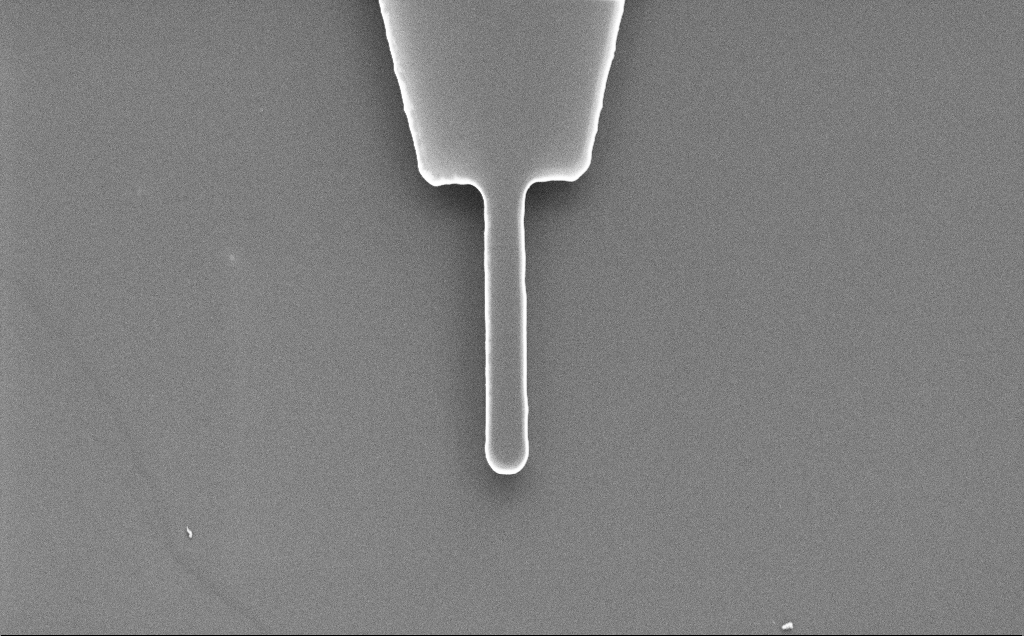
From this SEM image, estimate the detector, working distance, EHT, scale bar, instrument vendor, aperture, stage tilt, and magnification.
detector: SE2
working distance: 7 mm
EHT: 5 kV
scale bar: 10000 nm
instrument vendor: Zeiss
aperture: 30 µm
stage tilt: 0°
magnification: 3.01 K X